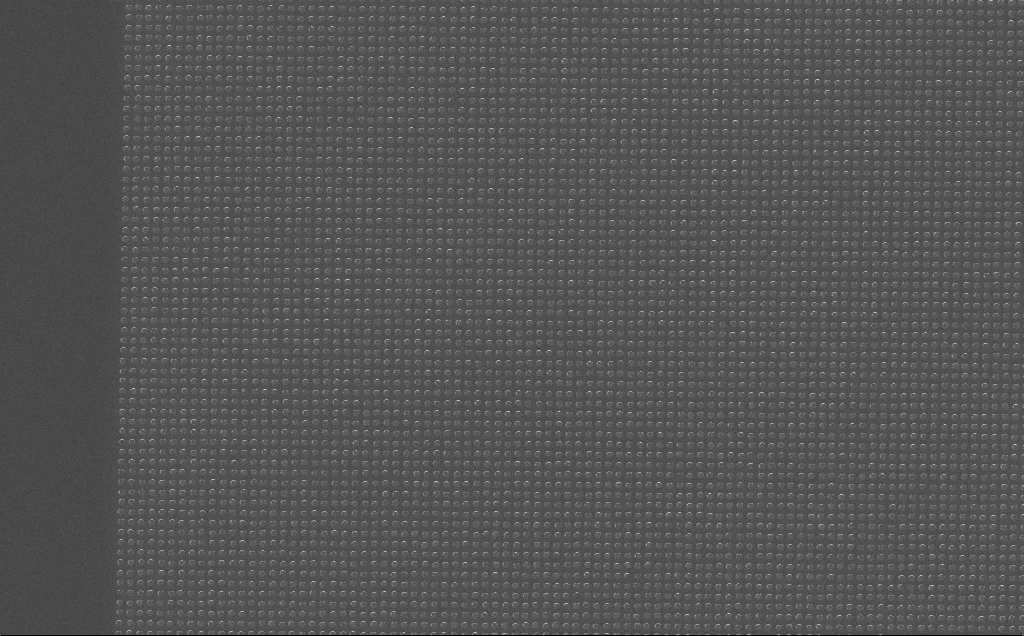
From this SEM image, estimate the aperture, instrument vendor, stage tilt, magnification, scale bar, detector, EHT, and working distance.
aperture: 30 µm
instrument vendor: Zeiss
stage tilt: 0°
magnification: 9.36 K X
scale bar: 2000 nm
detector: InLens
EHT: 10 kV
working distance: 7 mm